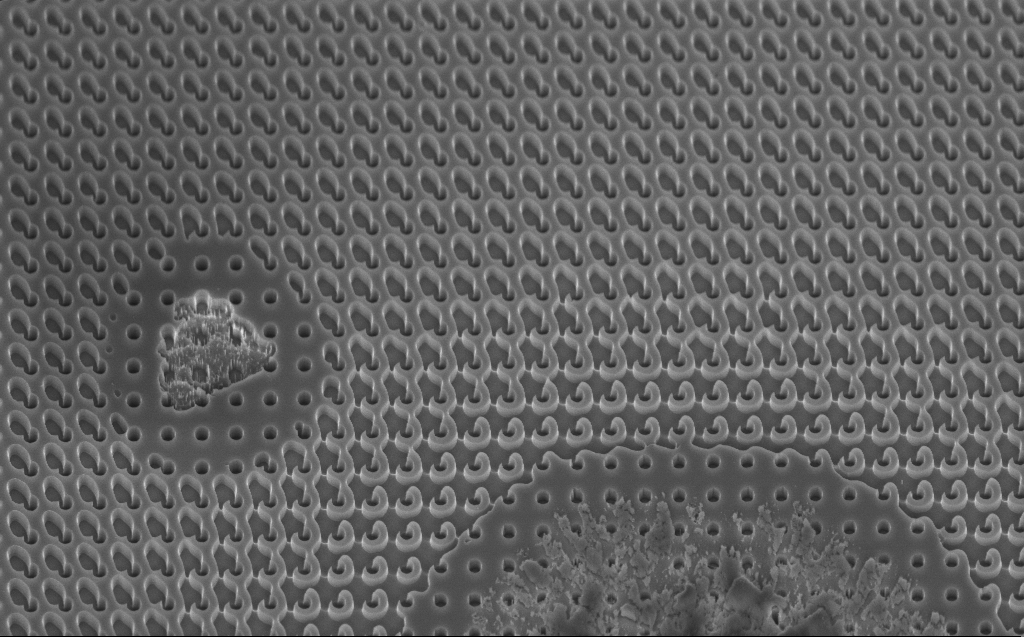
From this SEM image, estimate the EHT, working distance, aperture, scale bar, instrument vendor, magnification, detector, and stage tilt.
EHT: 5 kV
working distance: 6 mm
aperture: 30 µm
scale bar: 1000 nm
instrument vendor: Zeiss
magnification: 11.97 K X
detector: InLens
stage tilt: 45°